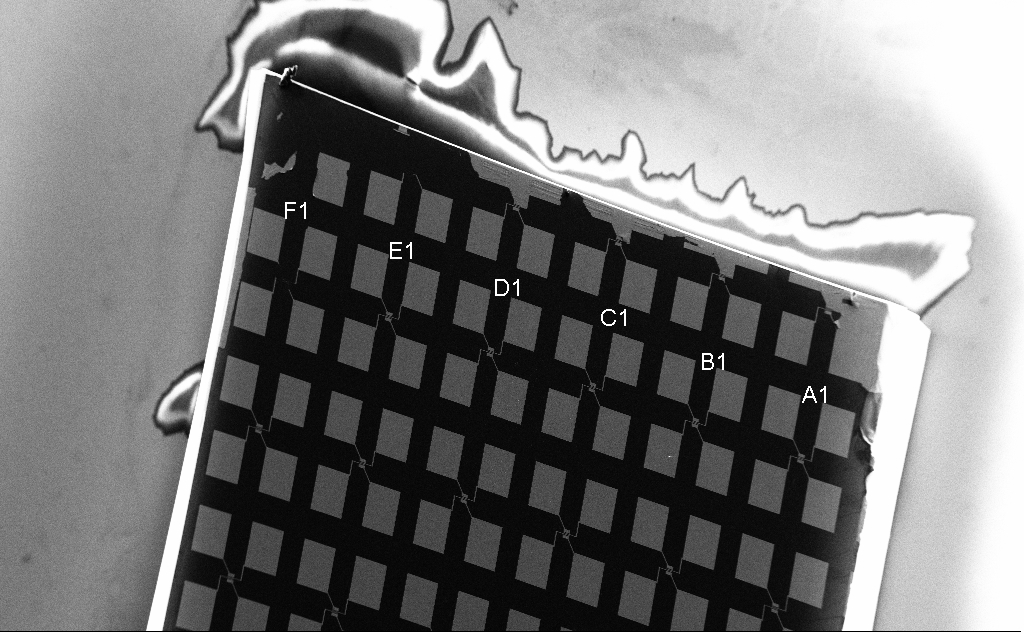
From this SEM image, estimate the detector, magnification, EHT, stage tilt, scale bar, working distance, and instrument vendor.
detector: SE2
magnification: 0.083 K X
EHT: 5 kV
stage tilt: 0°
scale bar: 200000 nm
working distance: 5 mm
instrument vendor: Zeiss